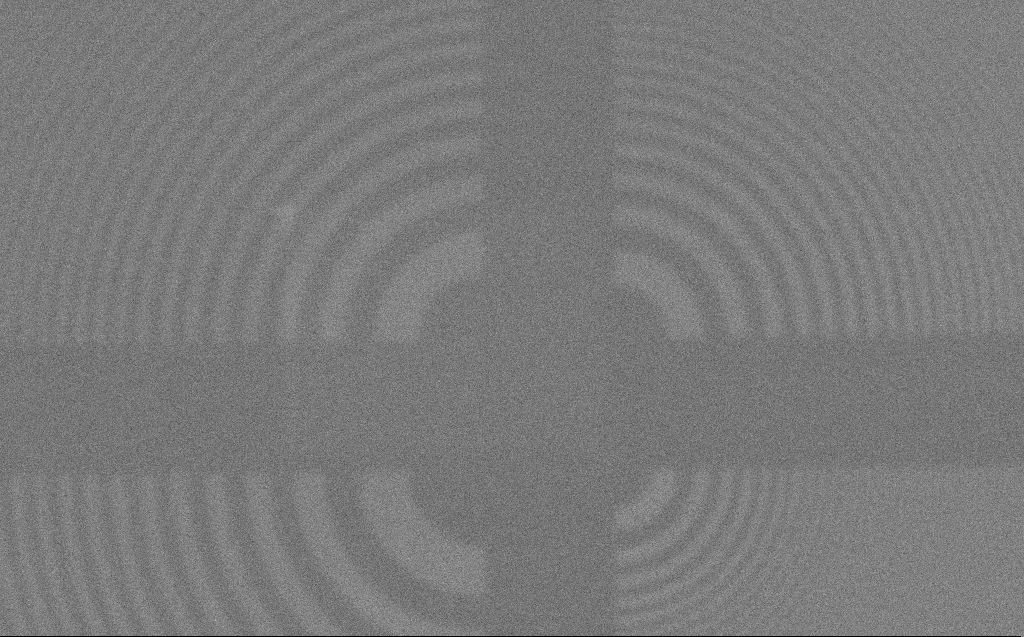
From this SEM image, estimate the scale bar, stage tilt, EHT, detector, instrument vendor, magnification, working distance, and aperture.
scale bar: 10000 nm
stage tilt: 0°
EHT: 3 kV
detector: SE2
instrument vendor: Zeiss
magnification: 4.86 K X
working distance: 4 mm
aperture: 30 µm